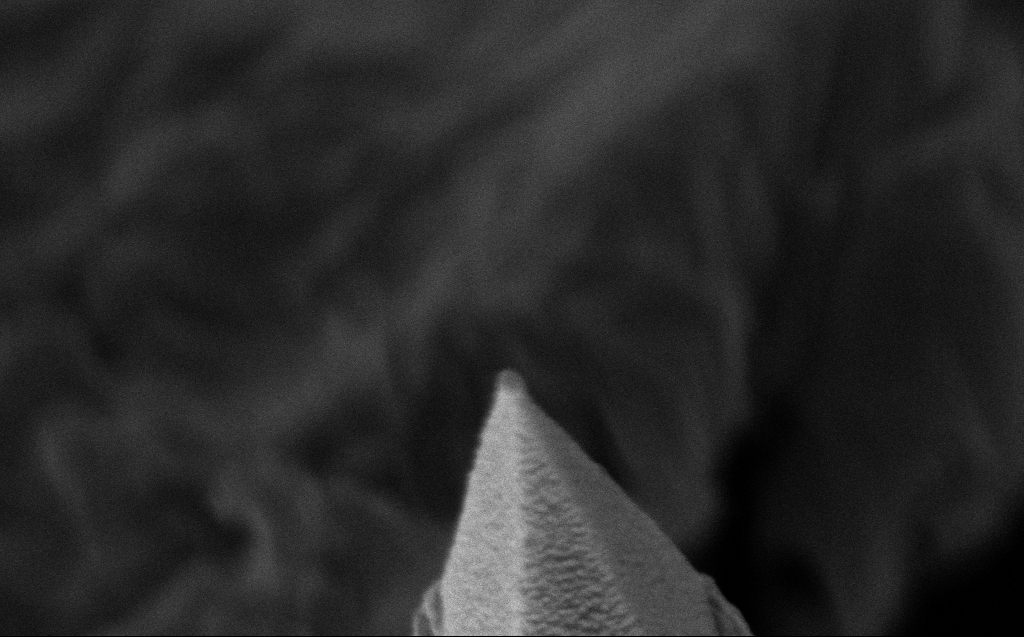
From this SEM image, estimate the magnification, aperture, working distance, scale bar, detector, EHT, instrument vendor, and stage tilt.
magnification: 121.6 K X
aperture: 30 µm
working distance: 7 mm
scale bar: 100 nm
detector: InLens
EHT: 4 kV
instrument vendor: Zeiss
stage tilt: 24.8°